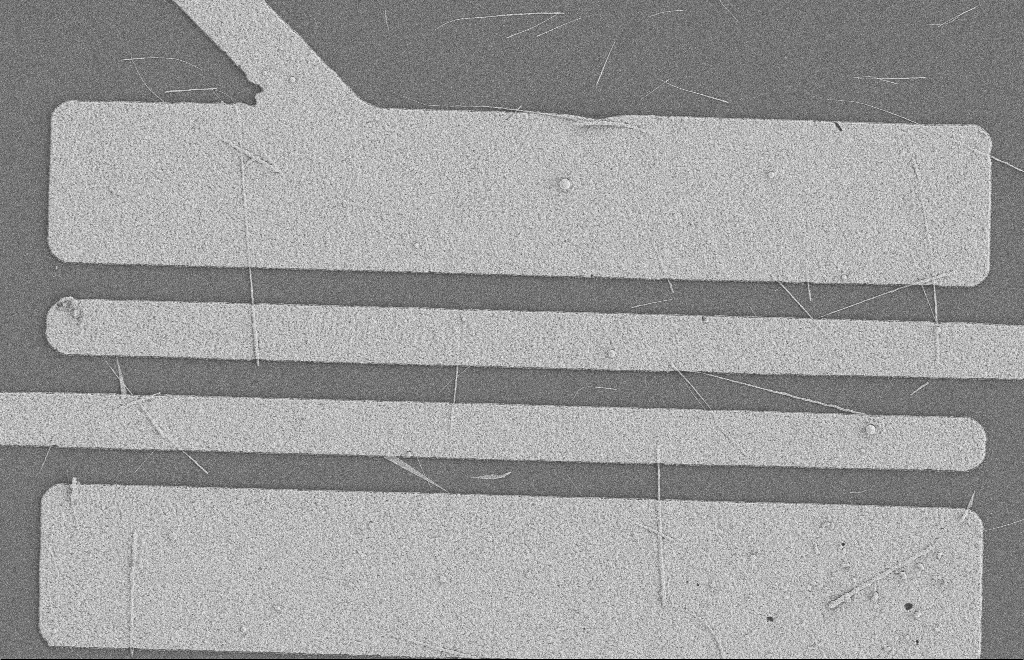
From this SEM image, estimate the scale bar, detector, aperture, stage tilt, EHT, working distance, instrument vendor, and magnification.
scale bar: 2000 nm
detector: SE2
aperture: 20 µm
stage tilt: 0°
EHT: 2 kV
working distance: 8 mm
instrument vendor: Zeiss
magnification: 5.65 K X